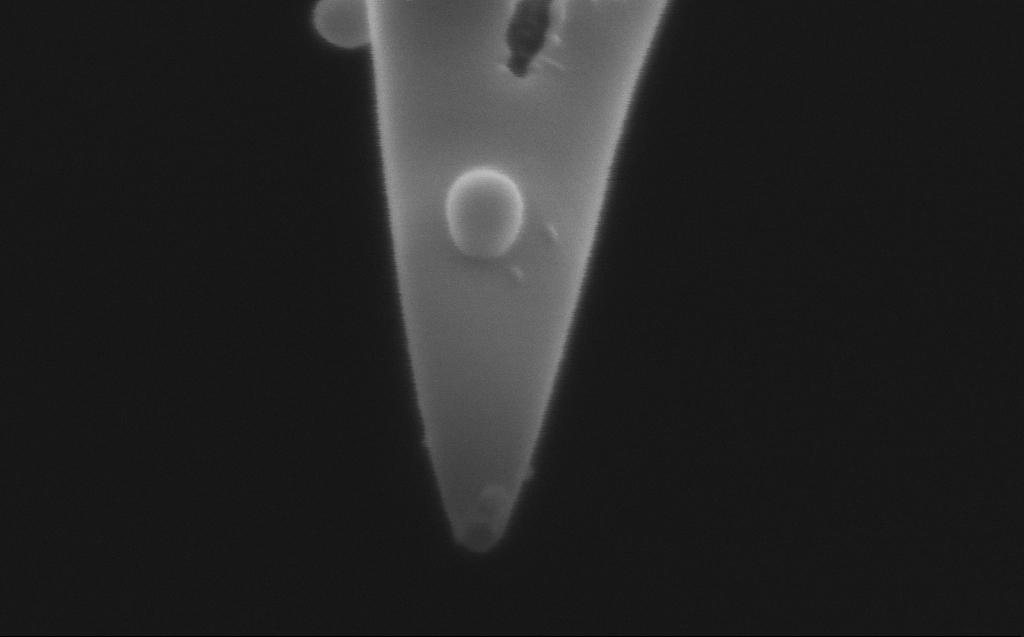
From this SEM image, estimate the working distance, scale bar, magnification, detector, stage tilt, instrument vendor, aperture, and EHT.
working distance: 3 mm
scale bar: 200 nm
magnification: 267.98 K X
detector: InLens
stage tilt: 45.1°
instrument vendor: Zeiss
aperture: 20 µm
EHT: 2 kV